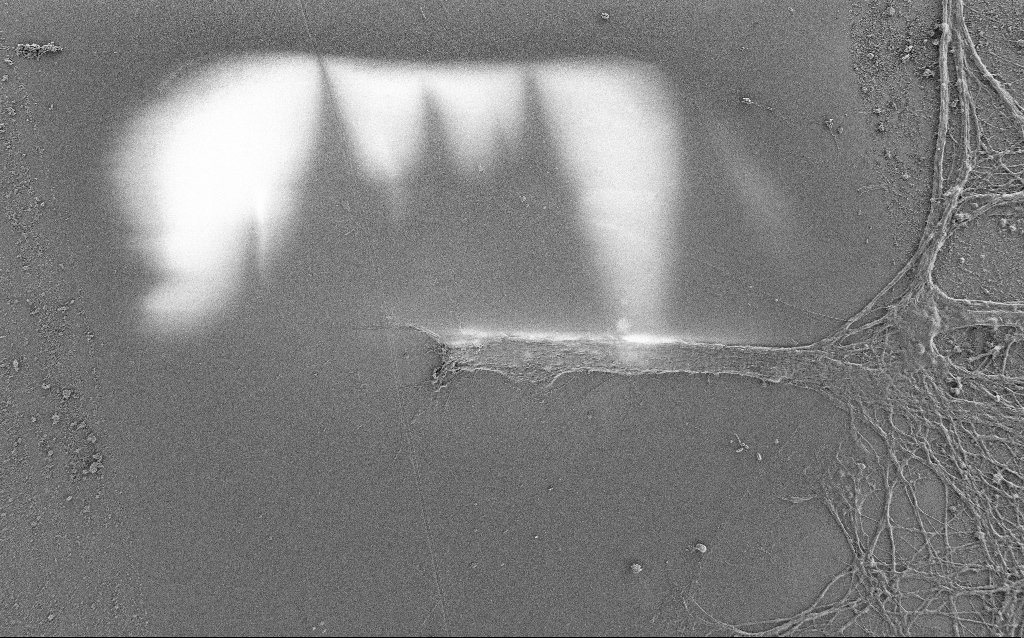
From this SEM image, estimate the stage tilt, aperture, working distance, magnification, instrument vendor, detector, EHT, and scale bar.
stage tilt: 0°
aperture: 30 µm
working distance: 4 mm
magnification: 1 K X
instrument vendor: Zeiss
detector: SE2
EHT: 0.9 kV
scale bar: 20000 nm